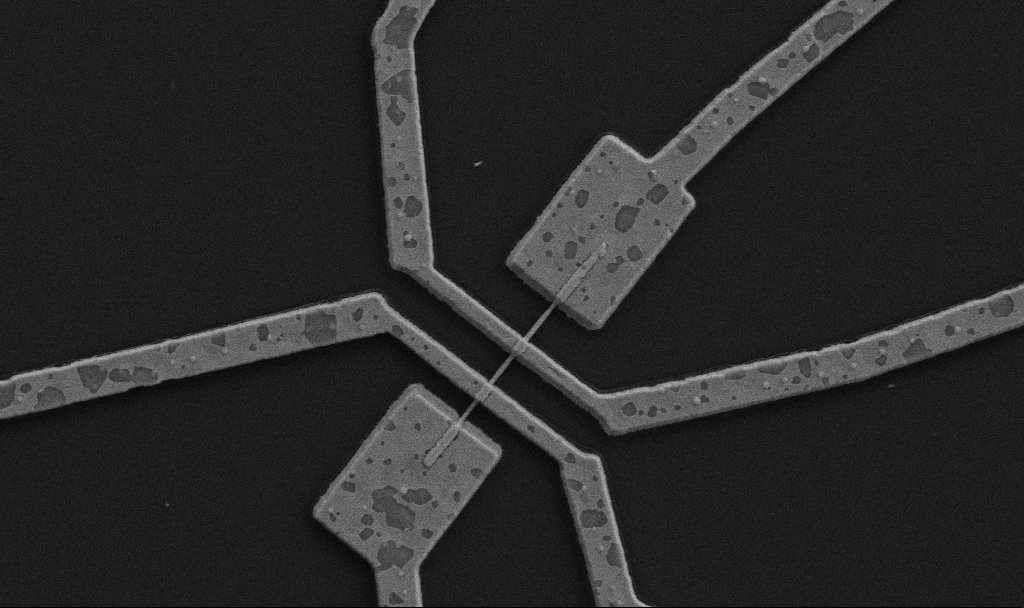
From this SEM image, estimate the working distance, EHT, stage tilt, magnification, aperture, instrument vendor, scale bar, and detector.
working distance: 10.7 mm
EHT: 5 kV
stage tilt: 0°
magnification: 20 K X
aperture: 30 µm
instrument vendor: Zeiss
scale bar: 1000 nm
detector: SE2